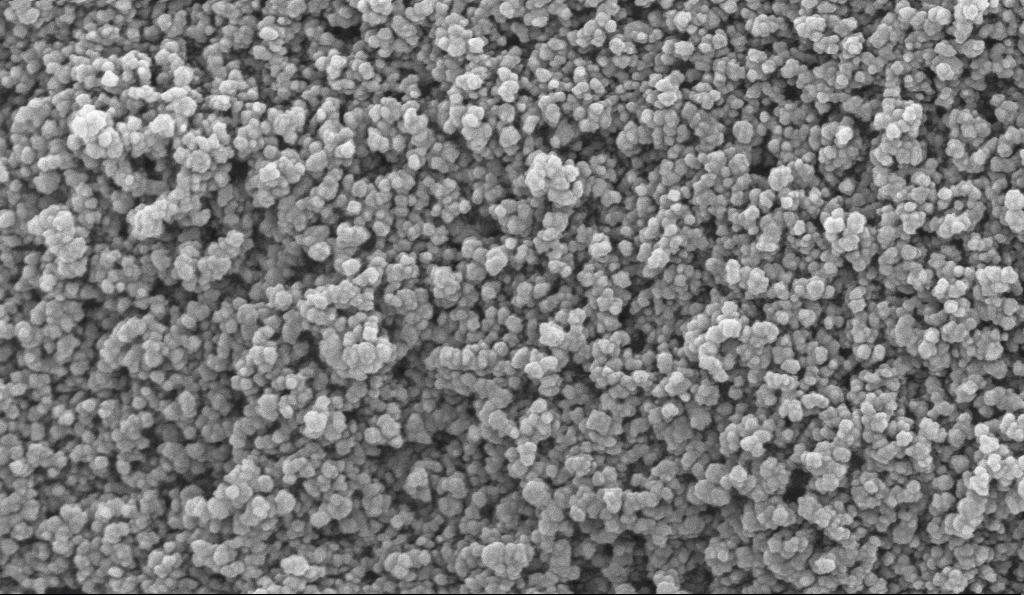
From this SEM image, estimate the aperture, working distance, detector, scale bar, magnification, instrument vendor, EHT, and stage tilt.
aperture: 30 µm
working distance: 5.1 mm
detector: InLens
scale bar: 100 nm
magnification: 135 K X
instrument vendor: Zeiss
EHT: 10 kV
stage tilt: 0°